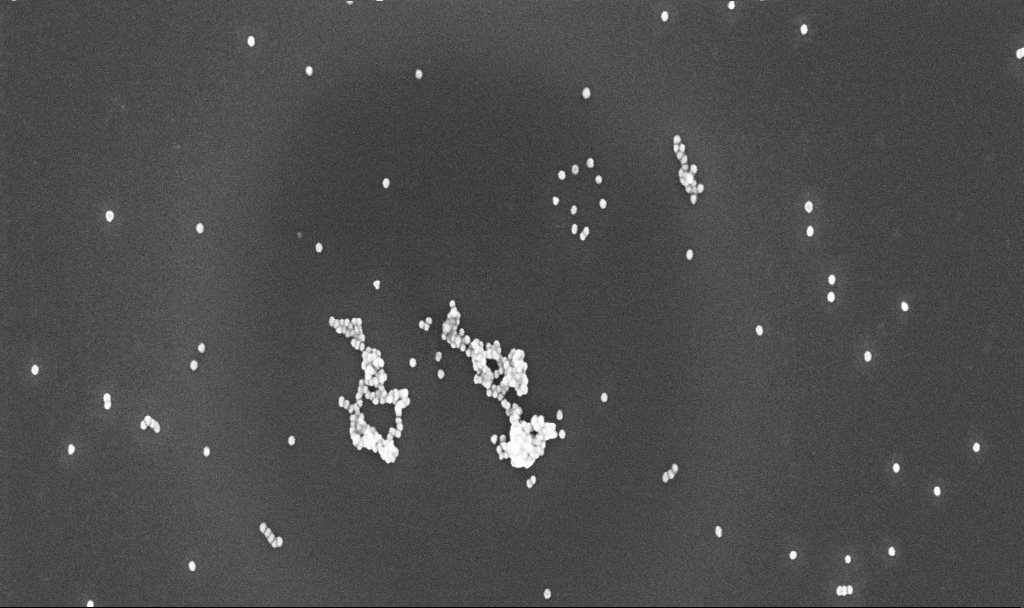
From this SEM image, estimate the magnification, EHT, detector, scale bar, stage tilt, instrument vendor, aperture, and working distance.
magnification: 100 K X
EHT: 10 kV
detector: InLens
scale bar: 200 nm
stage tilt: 0°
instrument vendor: Zeiss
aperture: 30 µm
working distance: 4.8 mm